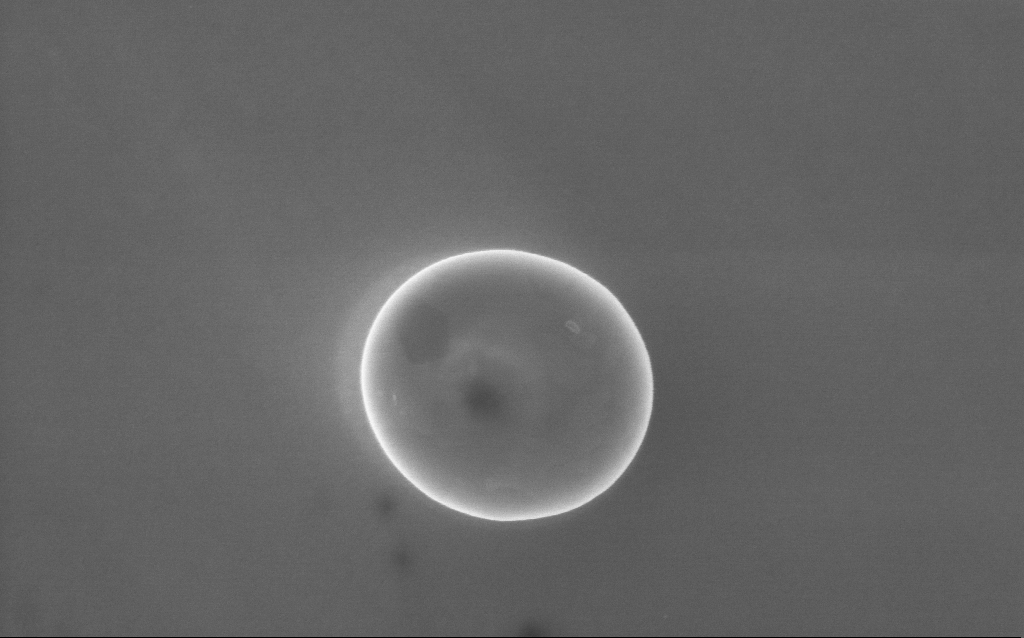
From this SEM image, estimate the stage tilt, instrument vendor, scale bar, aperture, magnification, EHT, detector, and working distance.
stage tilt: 0°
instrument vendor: Zeiss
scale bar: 1000 nm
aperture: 30 µm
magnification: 62 K X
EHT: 5 kV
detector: InLens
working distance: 3 mm